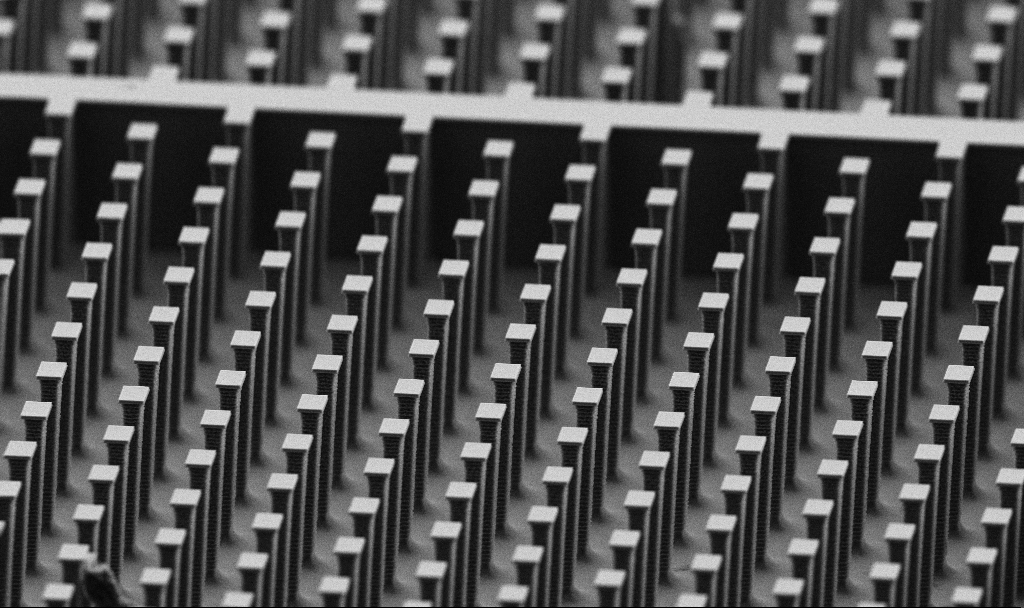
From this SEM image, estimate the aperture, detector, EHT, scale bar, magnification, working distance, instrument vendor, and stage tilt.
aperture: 30 µm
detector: SE2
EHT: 5 kV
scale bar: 10000 nm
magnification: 3.29 K X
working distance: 5.6 mm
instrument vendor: Zeiss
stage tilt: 70°